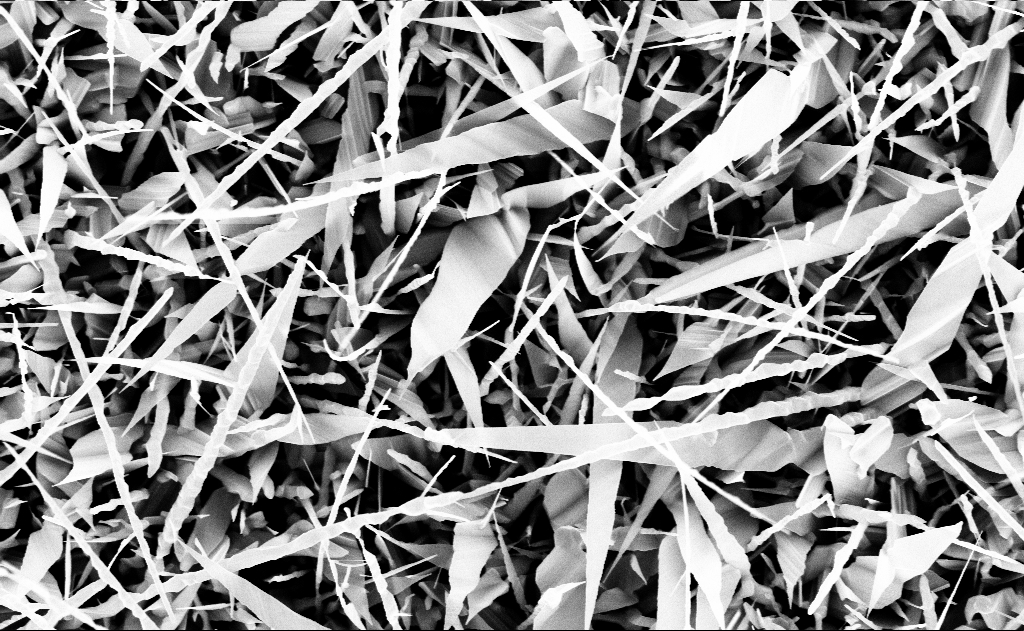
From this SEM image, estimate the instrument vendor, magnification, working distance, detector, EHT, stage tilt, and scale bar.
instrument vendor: Zeiss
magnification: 20 K X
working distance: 13 mm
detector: InLens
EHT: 10 kV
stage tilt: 0°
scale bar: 1000 nm